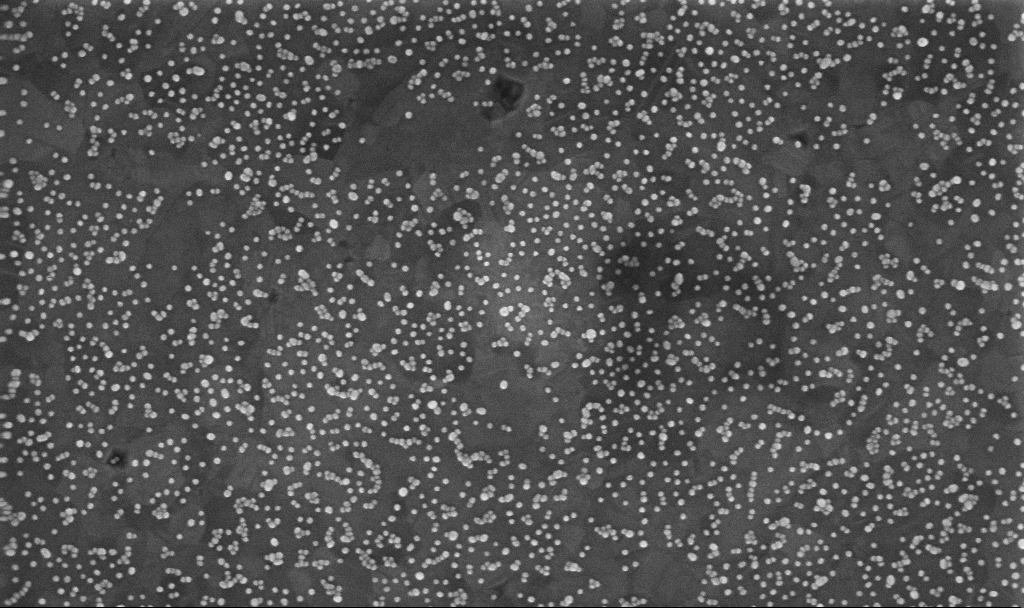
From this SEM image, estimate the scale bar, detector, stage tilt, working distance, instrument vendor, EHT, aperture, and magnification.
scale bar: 200 nm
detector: InLens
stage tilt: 0°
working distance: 3.4 mm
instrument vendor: Zeiss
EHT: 10 kV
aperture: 30 µm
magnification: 117.13 K X